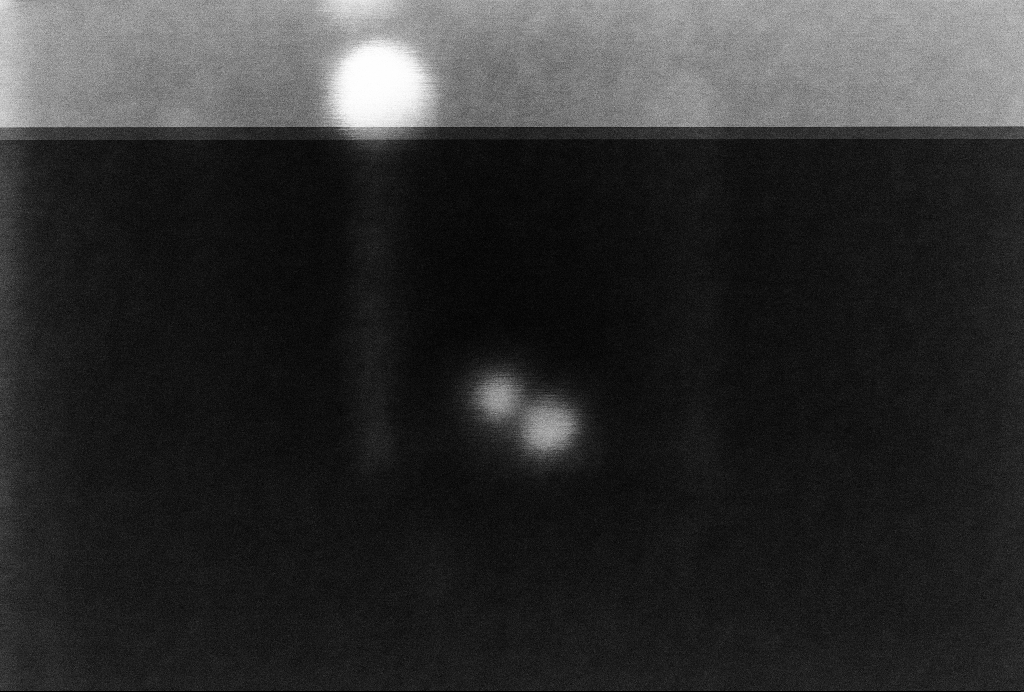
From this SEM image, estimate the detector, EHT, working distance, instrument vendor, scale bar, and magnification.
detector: InLens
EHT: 2 kV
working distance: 3.3 mm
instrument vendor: Zeiss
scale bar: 20 nm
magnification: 872.72 K X